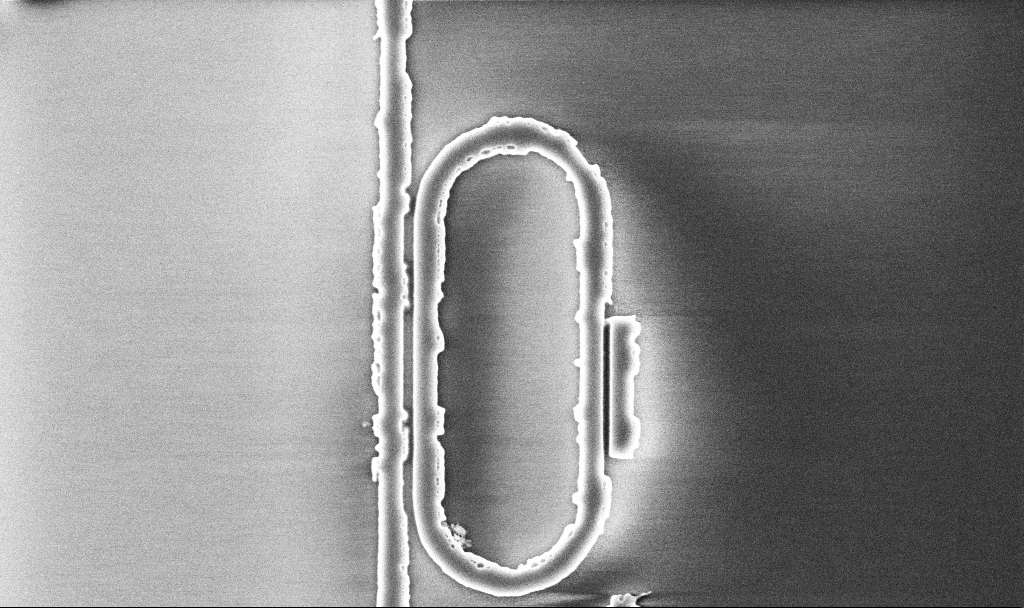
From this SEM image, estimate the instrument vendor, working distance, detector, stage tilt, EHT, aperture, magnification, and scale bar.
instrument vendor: Zeiss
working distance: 10.1 mm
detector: InLens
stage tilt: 0°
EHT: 5 kV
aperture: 30 µm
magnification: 17.62 K X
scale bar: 2000 nm